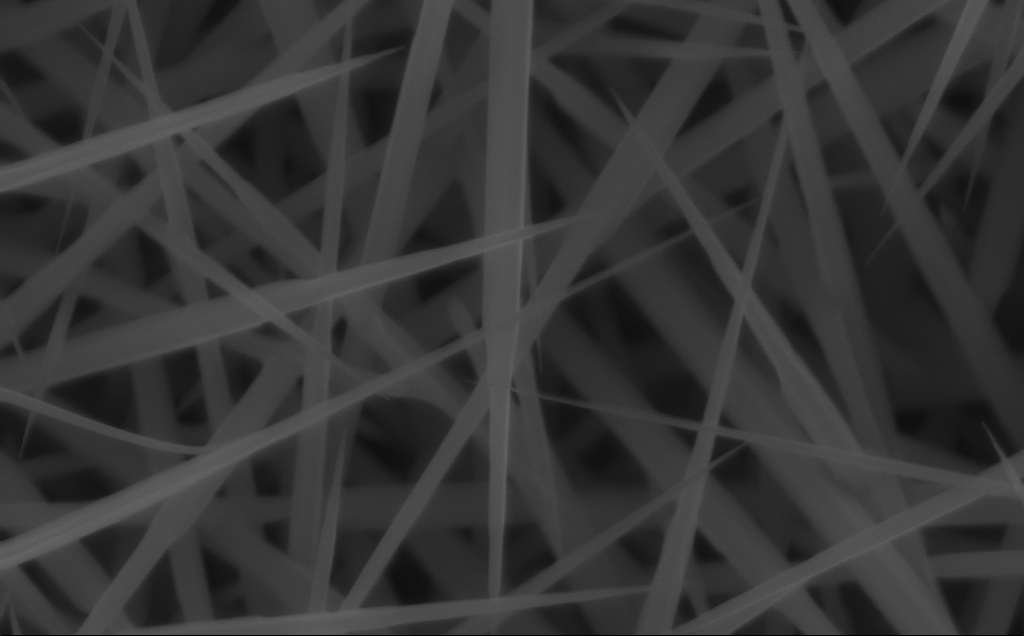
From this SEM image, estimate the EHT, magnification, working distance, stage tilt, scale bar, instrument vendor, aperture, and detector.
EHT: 10 kV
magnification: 150 K X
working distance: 4 mm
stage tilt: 0°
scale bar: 100 nm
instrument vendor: Zeiss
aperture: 30 µm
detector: InLens